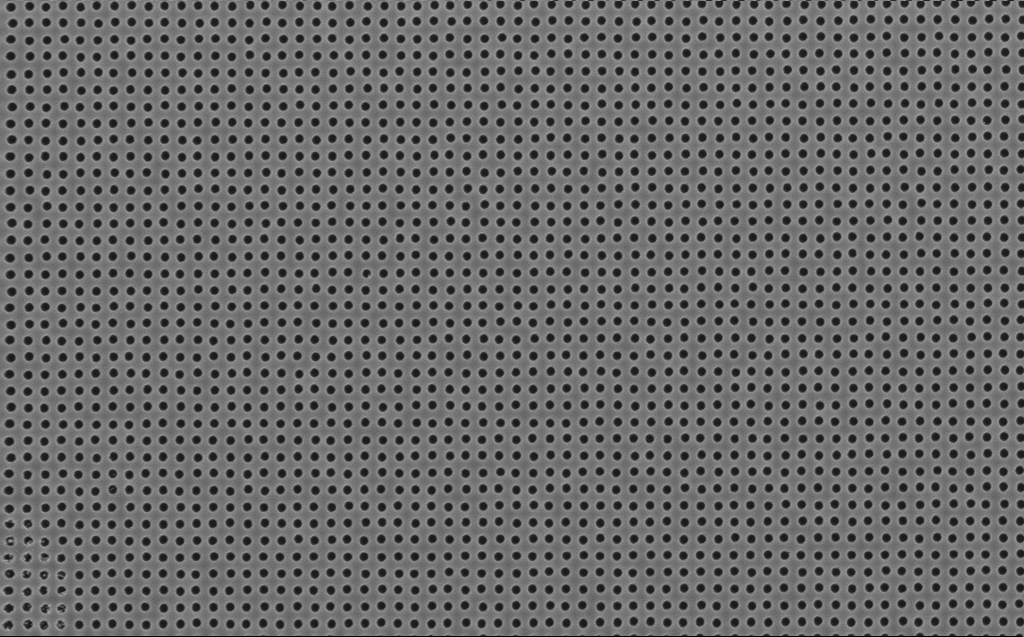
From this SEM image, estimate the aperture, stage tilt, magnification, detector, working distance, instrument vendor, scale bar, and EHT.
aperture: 30 µm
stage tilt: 0°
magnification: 15.44 K X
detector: InLens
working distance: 7 mm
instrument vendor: Zeiss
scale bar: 2000 nm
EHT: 5 kV